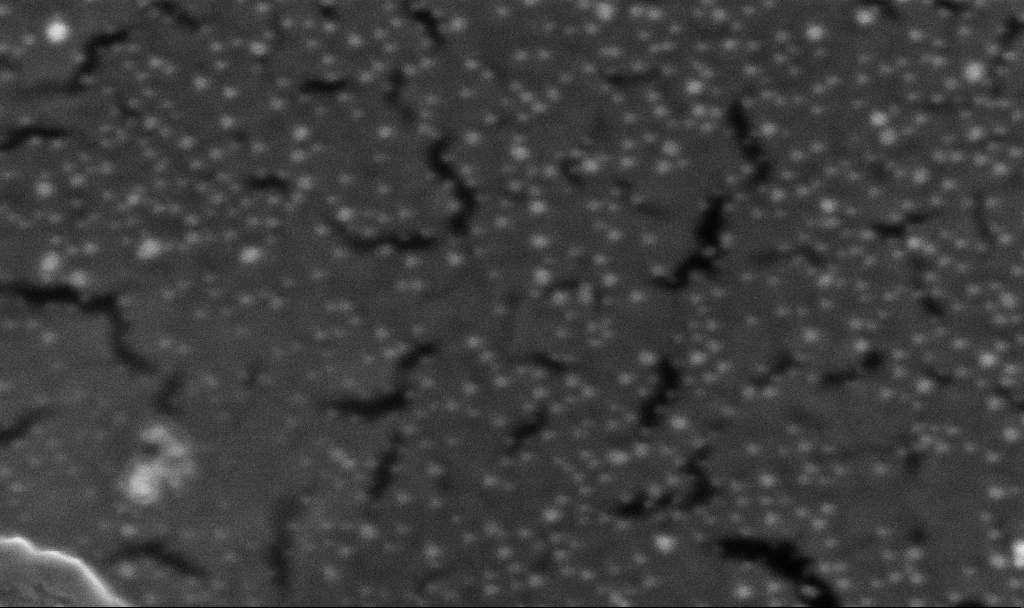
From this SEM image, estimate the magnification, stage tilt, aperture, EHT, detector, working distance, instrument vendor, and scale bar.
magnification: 245.9 K X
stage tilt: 0°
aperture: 30 µm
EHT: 5 kV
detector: InLens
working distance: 3.3 mm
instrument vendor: Zeiss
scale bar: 200 nm